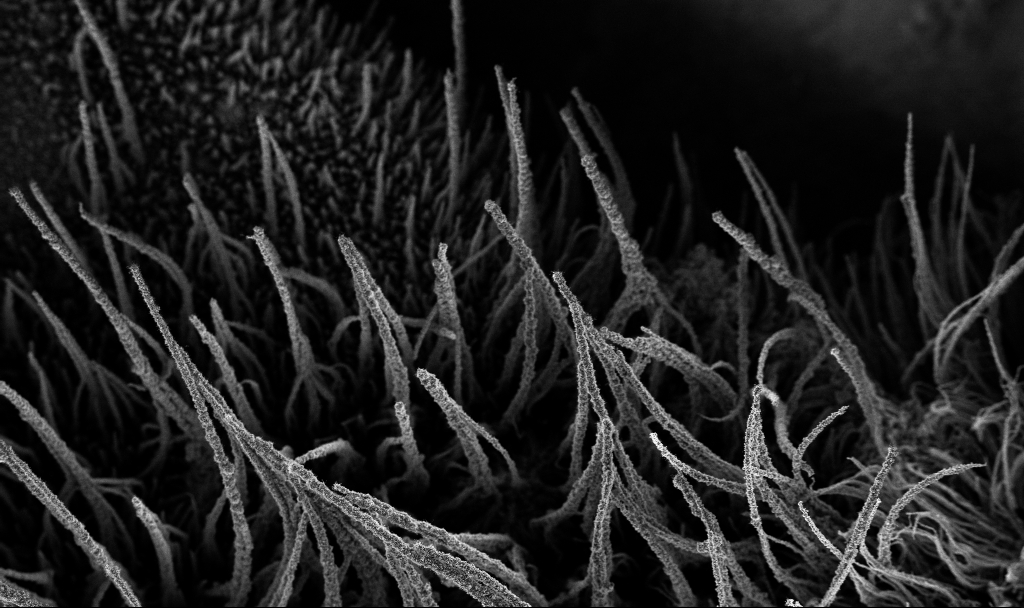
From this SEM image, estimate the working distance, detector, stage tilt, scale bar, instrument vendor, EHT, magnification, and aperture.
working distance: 3.4 mm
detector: InLens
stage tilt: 0°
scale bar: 100000 nm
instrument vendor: Zeiss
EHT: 3 kV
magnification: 0.25 K X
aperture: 30 µm